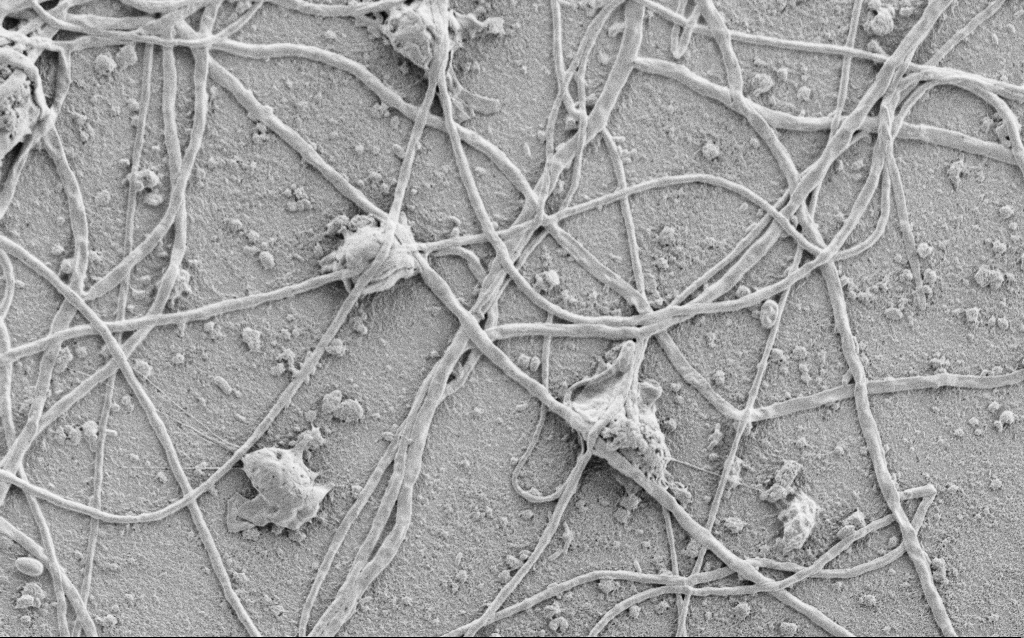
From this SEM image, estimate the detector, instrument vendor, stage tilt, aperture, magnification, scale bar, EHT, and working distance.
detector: SE2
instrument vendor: Zeiss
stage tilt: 0°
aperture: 30 µm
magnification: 2.5 K X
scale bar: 10000 nm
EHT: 1.5 kV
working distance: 6.8 mm